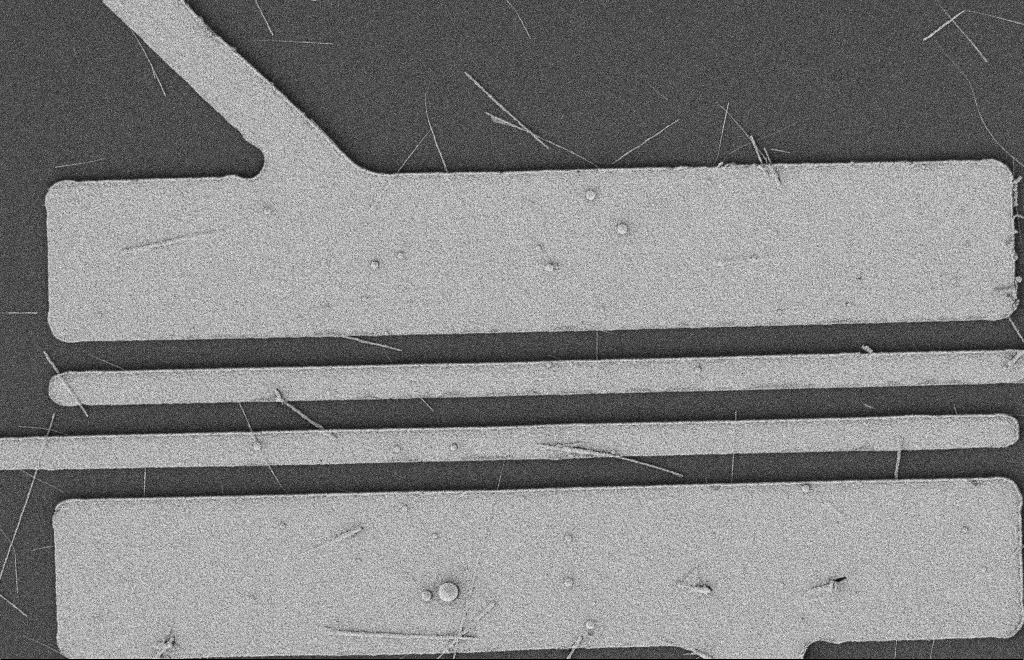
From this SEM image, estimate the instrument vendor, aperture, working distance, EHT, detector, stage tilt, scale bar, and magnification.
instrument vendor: Zeiss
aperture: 20 µm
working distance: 12 mm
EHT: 2 kV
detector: SE2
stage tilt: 0°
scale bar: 2000 nm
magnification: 5.79 K X